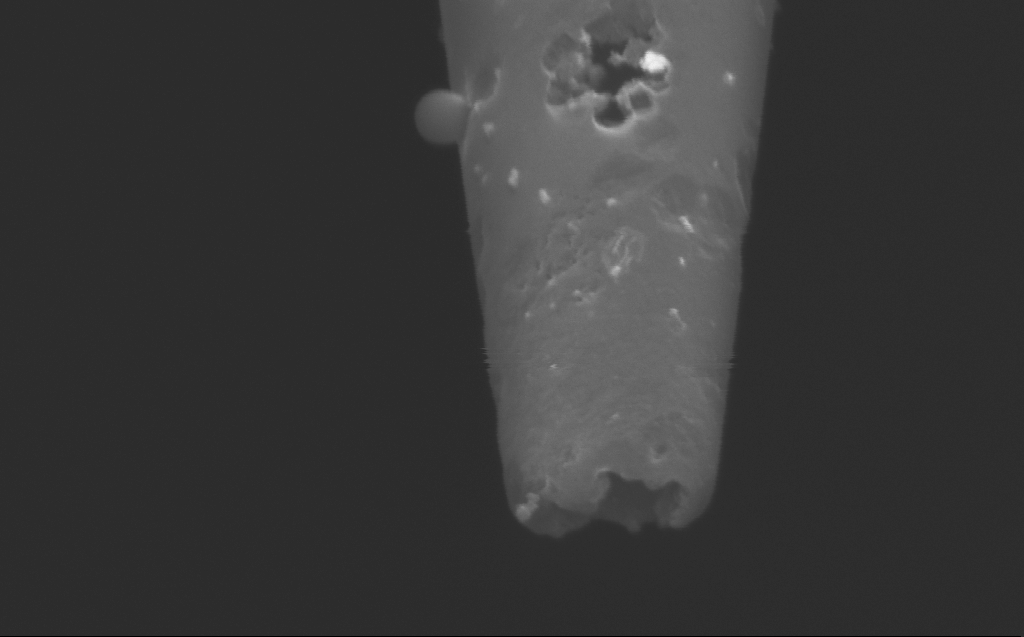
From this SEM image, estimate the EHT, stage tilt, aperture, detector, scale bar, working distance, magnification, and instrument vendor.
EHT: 5 kV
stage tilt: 45°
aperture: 30 µm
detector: InLens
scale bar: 200 nm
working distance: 4 mm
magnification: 172.57 K X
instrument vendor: Zeiss